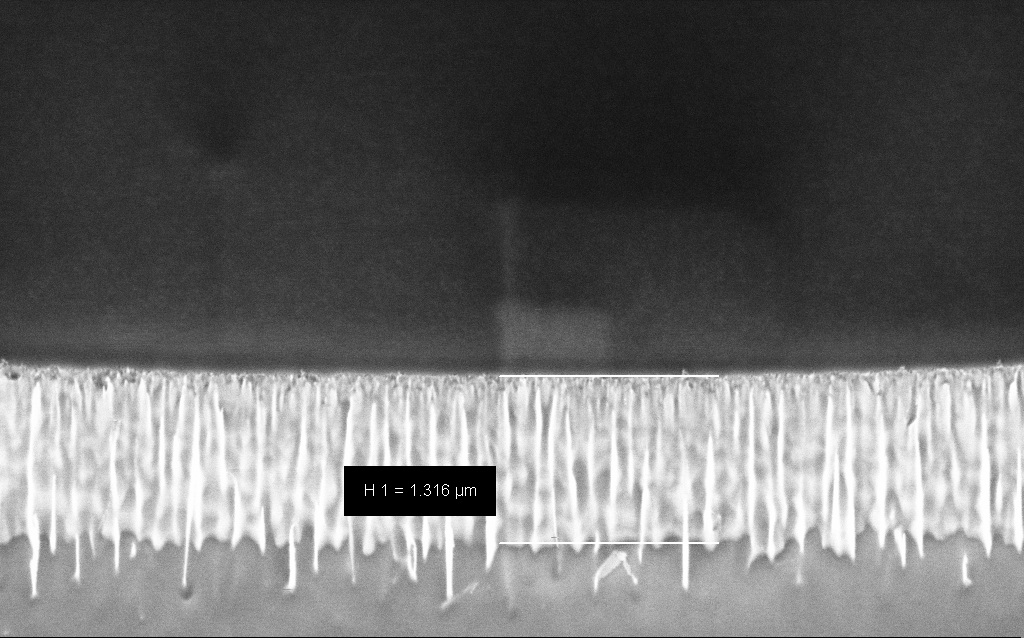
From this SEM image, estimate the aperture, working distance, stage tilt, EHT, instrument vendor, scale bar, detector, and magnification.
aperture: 30 µm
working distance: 6 mm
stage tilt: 45°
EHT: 3 kV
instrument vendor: Zeiss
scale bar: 1000 nm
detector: InLens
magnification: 46.59 K X